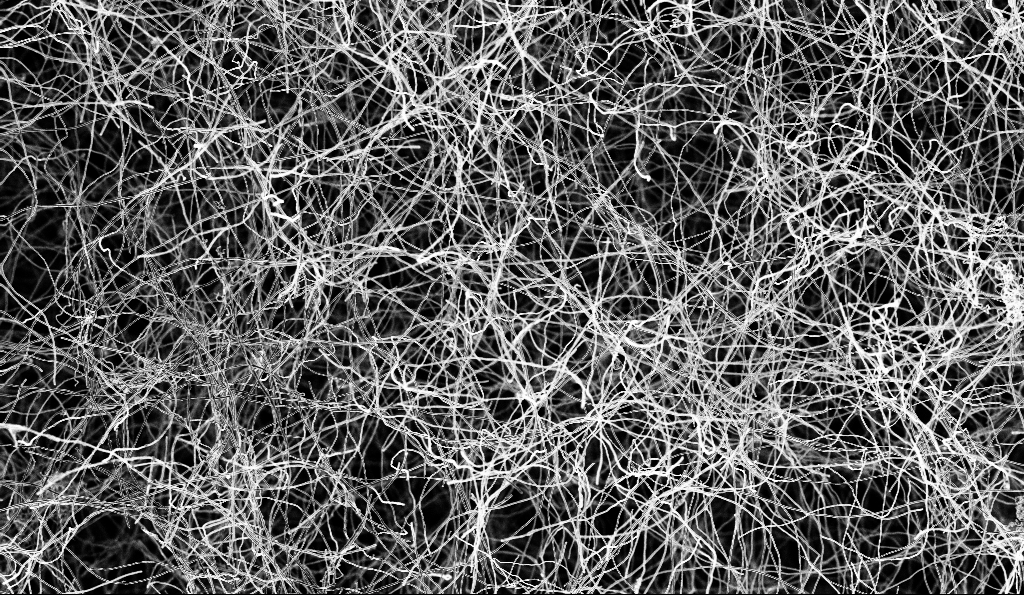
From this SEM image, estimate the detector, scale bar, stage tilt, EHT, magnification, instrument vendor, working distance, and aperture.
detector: InLens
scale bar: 20000 nm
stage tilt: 0°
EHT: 5 kV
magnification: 1 K X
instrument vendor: Zeiss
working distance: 4.8 mm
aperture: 30 µm